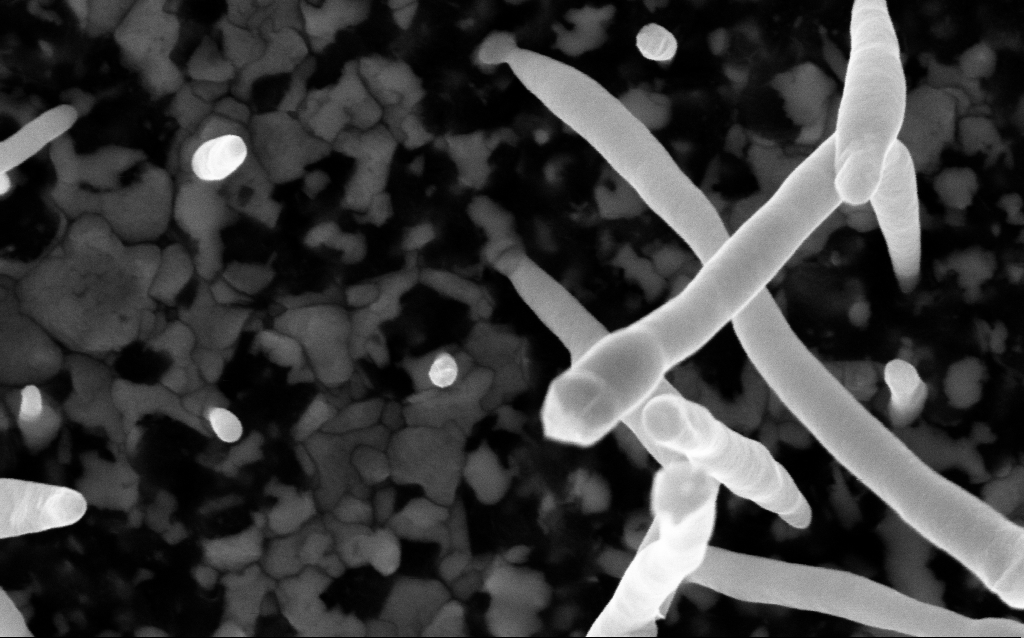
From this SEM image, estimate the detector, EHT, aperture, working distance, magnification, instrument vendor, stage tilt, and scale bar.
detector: InLens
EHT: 5 kV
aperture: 30 µm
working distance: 2.6 mm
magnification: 200 K X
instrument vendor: Zeiss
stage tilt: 0°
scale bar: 100 nm